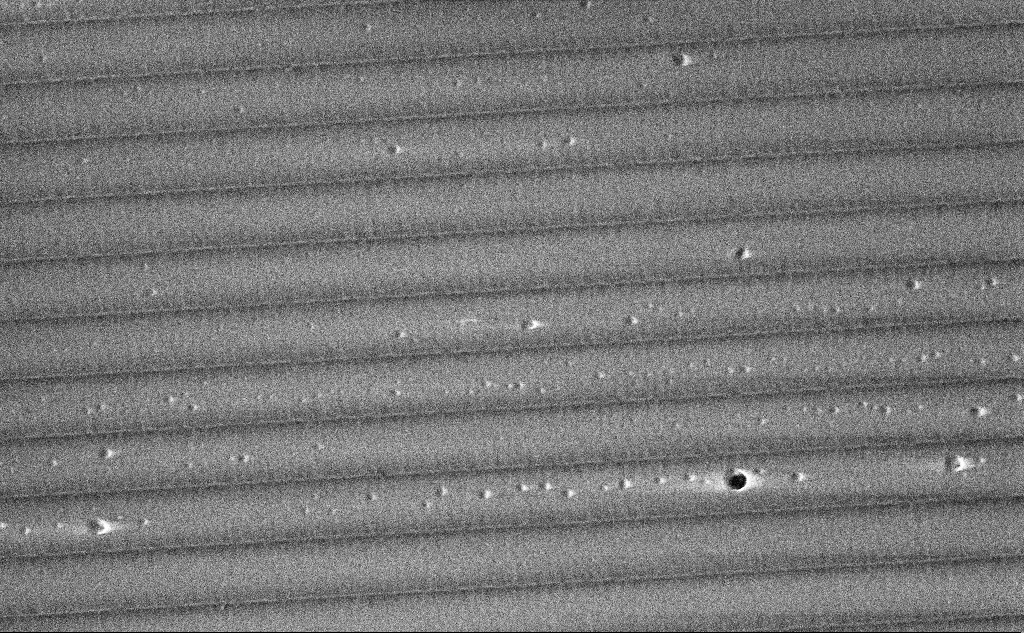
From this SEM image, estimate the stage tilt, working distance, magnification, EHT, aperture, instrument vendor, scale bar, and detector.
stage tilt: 0°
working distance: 6.3 mm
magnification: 10.9 K X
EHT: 3 kV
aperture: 30 µm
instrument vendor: Zeiss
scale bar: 2000 nm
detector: InLens